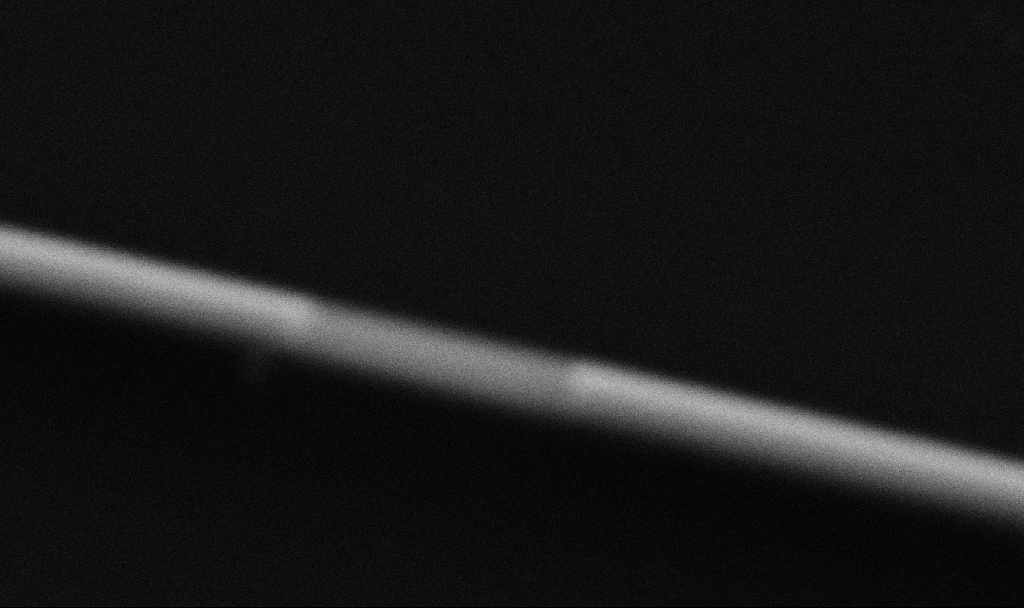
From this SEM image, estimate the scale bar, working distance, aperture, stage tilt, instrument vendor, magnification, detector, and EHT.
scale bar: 100 nm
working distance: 8.8 mm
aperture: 30 µm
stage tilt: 0°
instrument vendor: Zeiss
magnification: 300 K X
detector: SE2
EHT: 5 kV